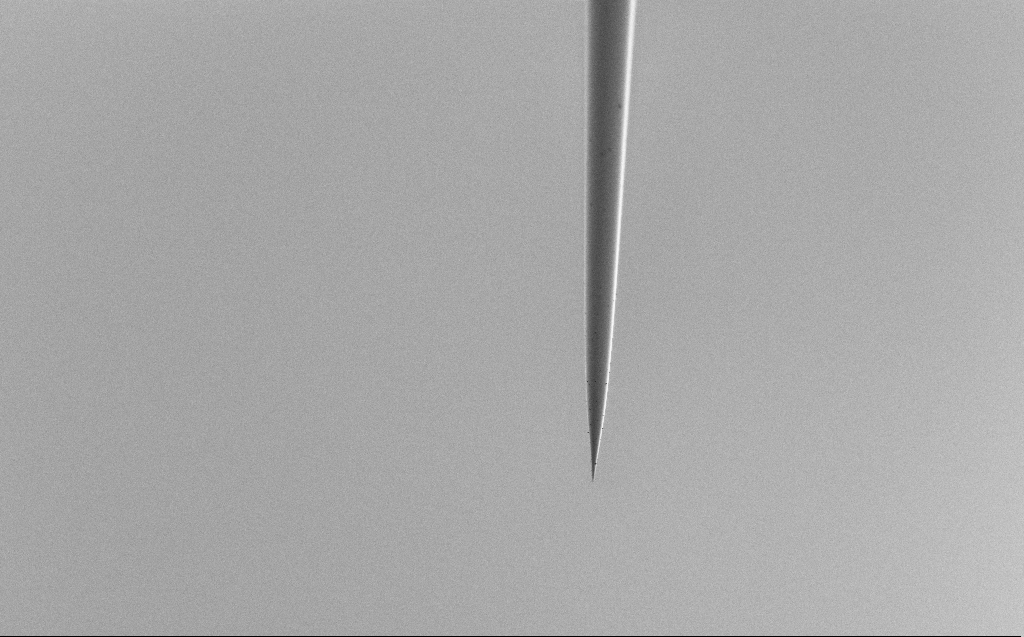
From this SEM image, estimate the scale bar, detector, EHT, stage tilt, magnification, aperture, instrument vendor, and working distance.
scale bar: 20000 nm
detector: SE2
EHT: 1 kV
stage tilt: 45.1°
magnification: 0.802 K X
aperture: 30 µm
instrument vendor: Zeiss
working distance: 3 mm